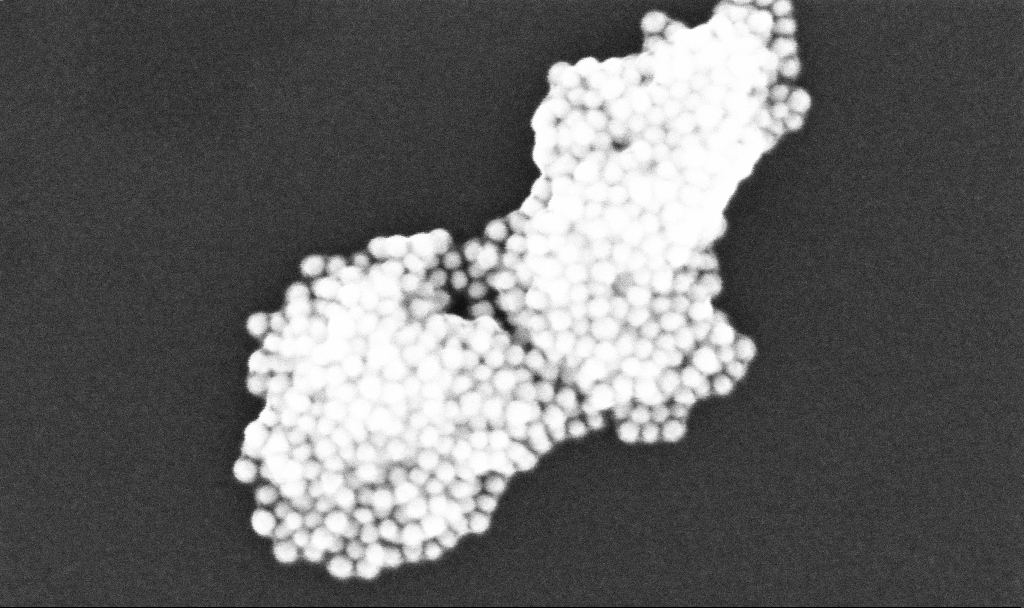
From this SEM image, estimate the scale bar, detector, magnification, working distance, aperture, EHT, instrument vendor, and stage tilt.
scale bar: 200 nm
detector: InLens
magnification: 361.96 K X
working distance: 3.3 mm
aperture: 30 µm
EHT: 10 kV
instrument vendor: Zeiss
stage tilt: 0°